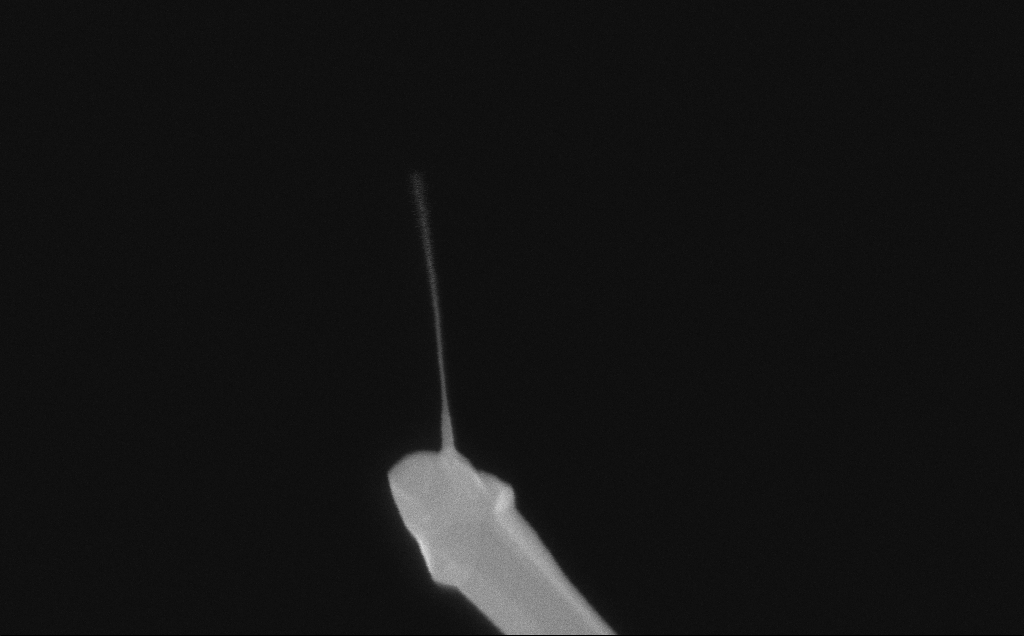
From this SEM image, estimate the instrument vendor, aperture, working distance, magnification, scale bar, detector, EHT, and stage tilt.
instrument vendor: Zeiss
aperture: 30 µm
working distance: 6 mm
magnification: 189.64 K X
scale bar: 200 nm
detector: InLens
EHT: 10 kV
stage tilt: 0°